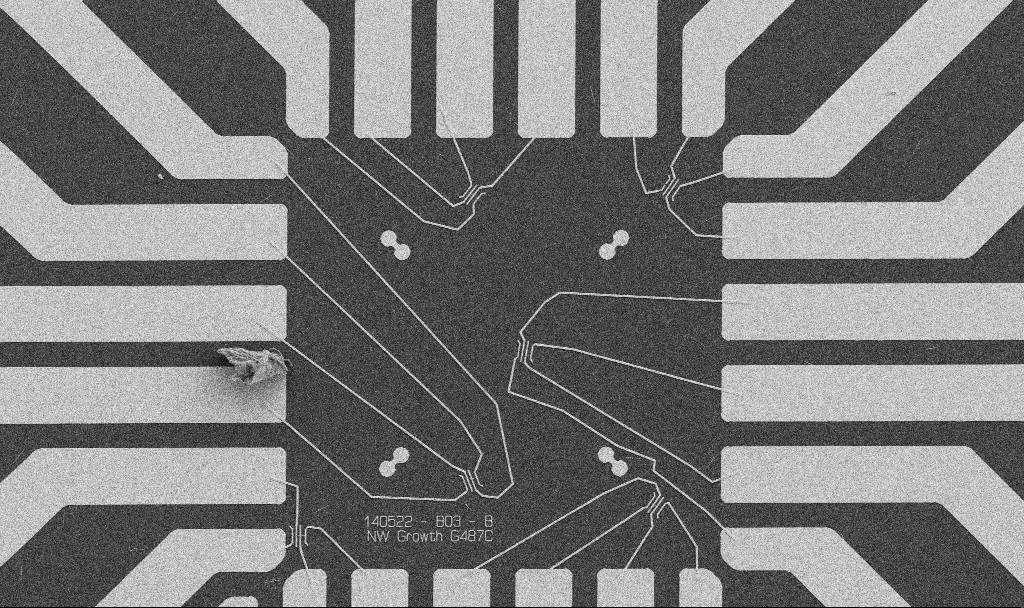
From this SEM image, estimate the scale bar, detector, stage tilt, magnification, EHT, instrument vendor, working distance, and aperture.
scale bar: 20000 nm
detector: SE2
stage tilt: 0°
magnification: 1 K X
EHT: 5 kV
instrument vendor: Zeiss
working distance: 10.7 mm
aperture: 30 µm